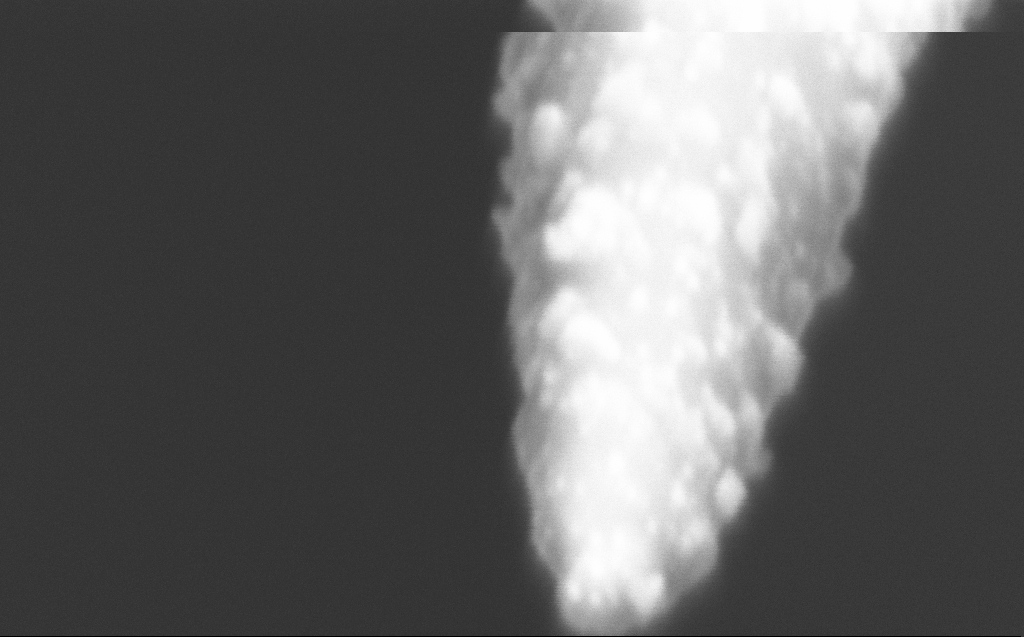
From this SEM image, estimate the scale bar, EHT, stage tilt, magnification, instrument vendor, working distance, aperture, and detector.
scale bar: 100 nm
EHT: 2 kV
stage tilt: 45°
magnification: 400 K X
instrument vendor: Zeiss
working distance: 6 mm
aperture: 30 µm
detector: InLens